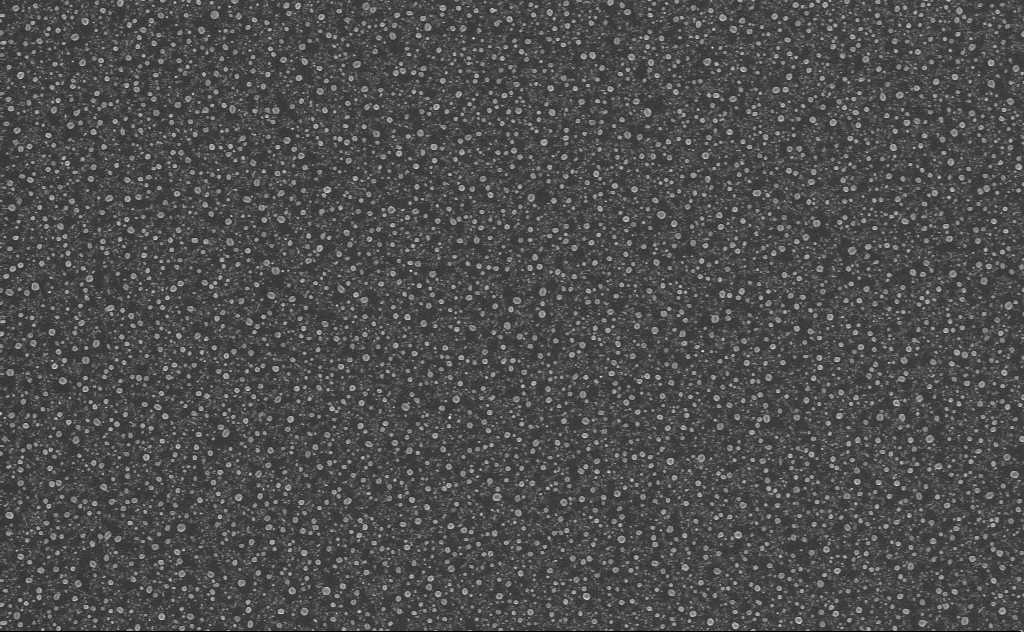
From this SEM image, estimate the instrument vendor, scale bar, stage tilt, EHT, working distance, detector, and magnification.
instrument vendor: Zeiss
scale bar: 2000 nm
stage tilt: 0°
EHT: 3 kV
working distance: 4 mm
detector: InLens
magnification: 20 K X